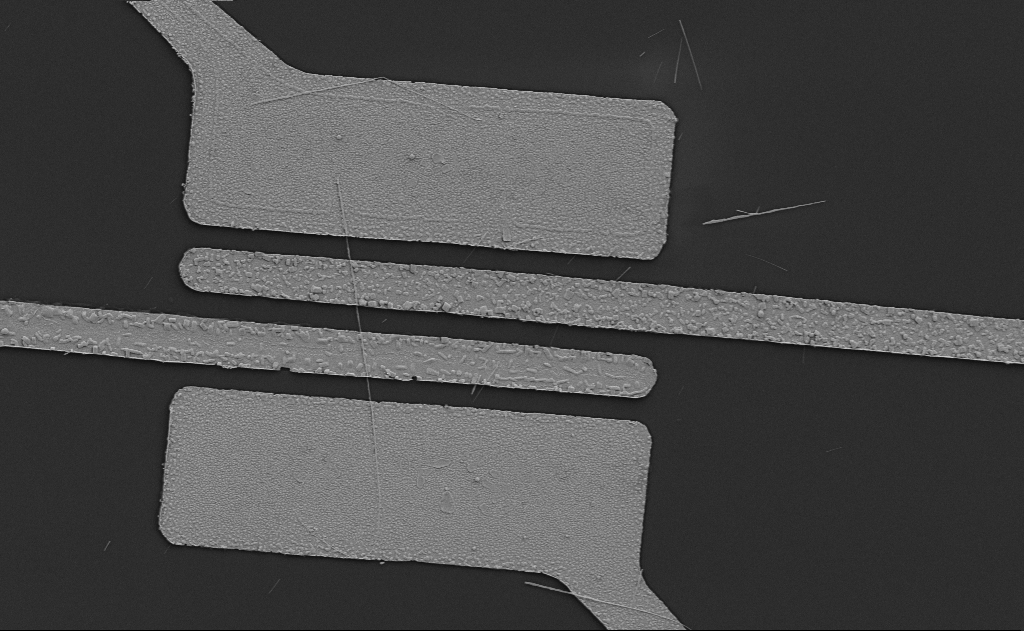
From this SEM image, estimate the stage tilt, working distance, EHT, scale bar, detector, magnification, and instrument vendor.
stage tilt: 0°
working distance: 13 mm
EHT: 5 kV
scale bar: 10000 nm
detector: SE2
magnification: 5.82 K X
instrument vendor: Zeiss